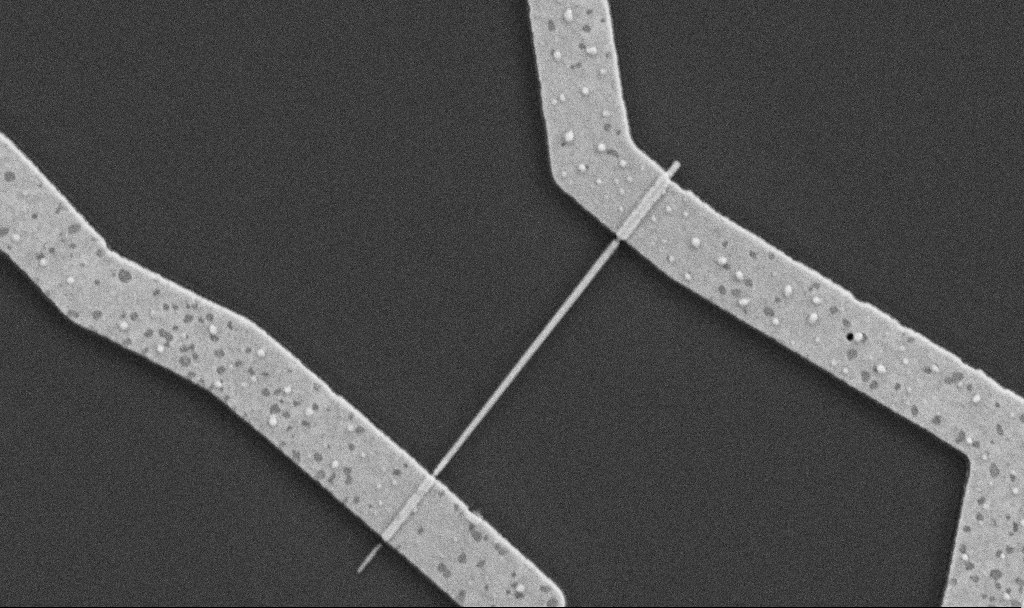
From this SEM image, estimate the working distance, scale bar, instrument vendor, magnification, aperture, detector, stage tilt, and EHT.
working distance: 8.7 mm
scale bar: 1000 nm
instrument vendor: Zeiss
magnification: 30 K X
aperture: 30 µm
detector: SE2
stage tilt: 0°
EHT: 5 kV